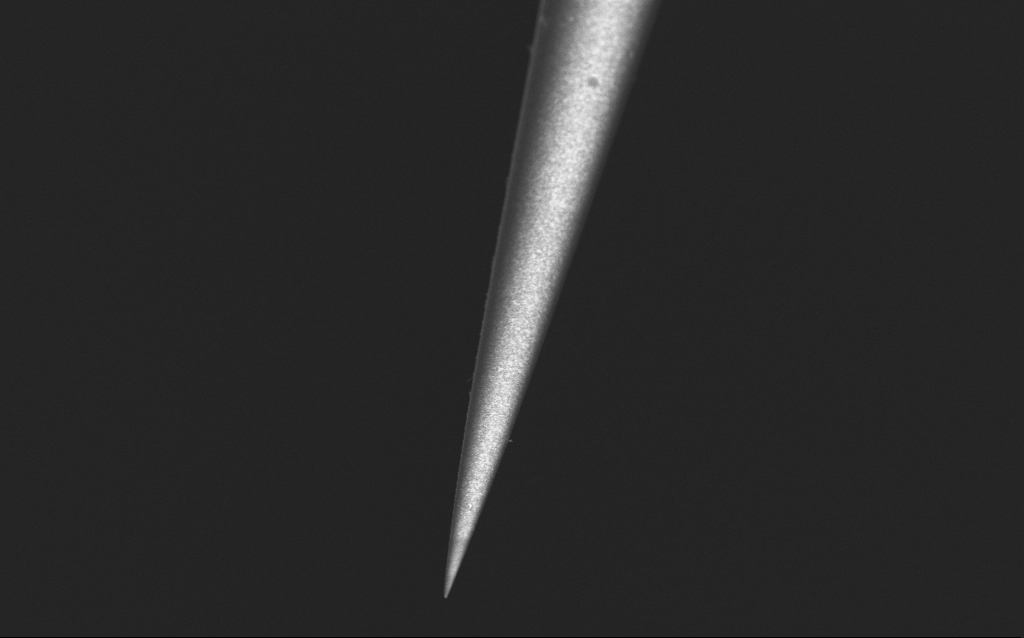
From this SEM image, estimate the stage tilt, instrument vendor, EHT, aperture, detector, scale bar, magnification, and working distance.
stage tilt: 45°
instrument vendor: Zeiss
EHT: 2.5 kV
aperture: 30 µm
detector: InLens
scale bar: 2000 nm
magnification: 10 K X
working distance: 5 mm